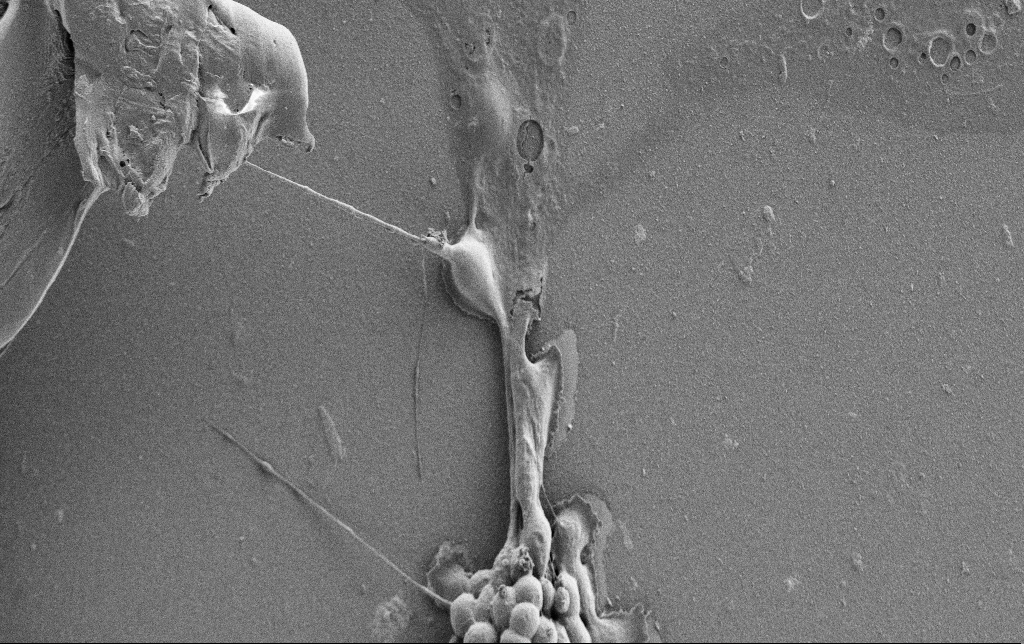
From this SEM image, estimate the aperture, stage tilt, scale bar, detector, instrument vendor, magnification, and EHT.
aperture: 30 µm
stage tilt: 0°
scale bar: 20000 nm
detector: SE2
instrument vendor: Zeiss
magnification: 2.5 K X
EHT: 0.9 kV